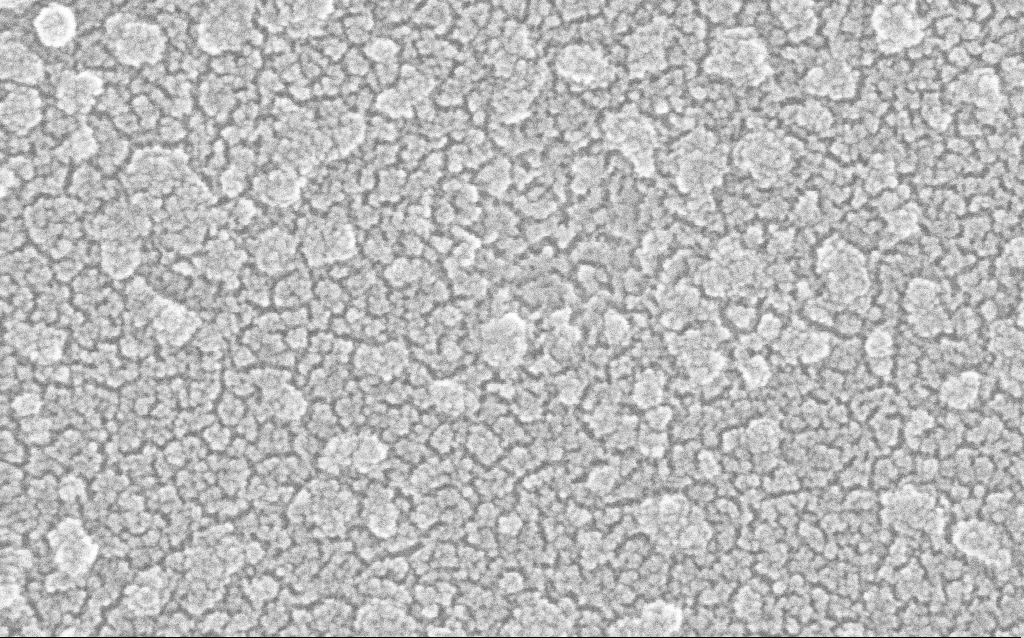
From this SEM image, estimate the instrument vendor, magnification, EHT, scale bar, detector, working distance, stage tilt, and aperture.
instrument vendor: Zeiss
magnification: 500 K X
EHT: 20 kV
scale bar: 100 nm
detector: InLens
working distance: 1.8 mm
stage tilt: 0°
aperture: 30 µm